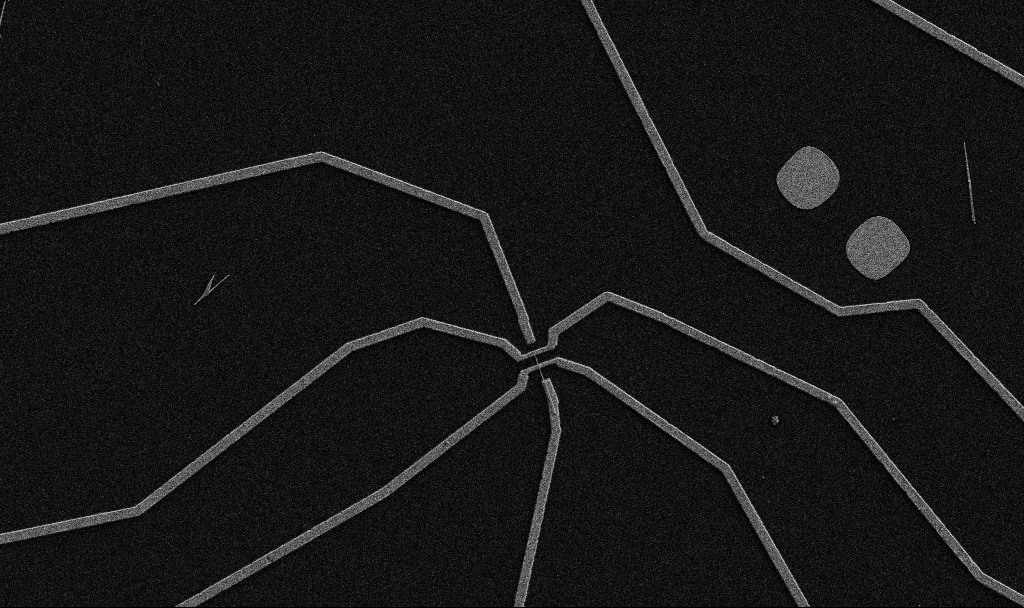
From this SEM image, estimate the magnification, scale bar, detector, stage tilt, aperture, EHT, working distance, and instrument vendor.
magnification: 5 K X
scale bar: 10000 nm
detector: SE2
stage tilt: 0°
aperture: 30 µm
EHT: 5 kV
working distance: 10.7 mm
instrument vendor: Zeiss